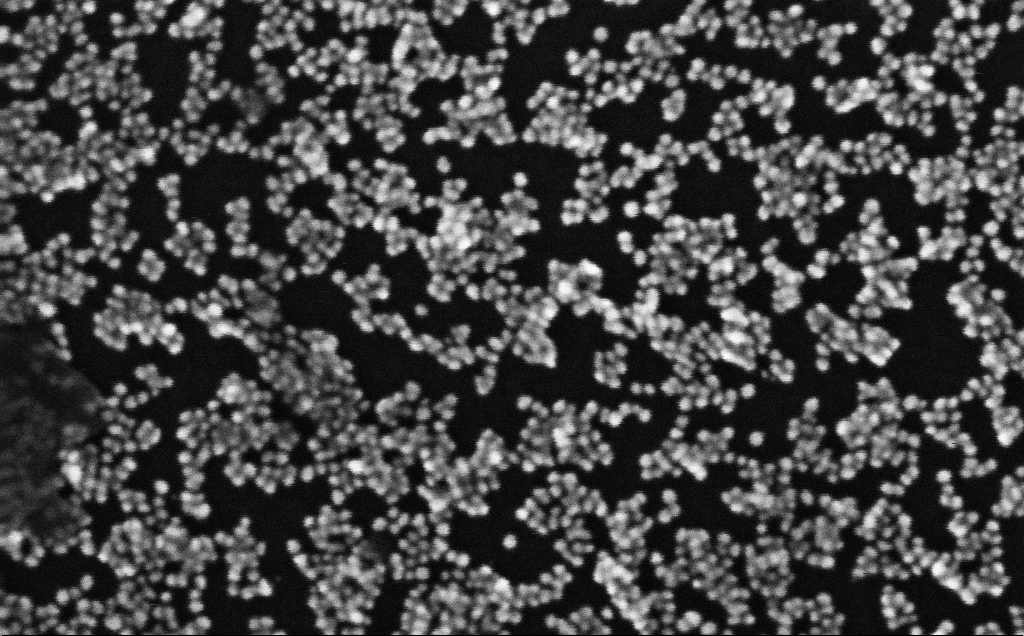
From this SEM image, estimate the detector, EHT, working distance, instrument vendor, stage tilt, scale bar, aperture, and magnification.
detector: InLens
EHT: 10 kV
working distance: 4.1 mm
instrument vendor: Zeiss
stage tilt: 0°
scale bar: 100 nm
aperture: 30 µm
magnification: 557.33 K X